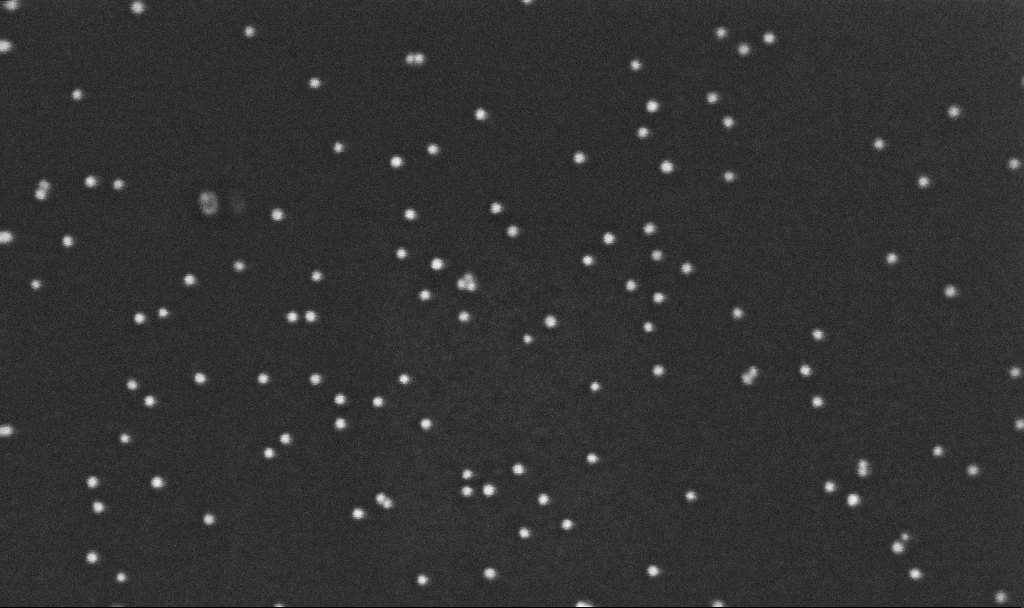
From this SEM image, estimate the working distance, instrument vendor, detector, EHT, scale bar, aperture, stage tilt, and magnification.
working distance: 3.2 mm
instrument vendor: Zeiss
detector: InLens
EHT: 10 kV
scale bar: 100 nm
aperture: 30 µm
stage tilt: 0°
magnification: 350 K X